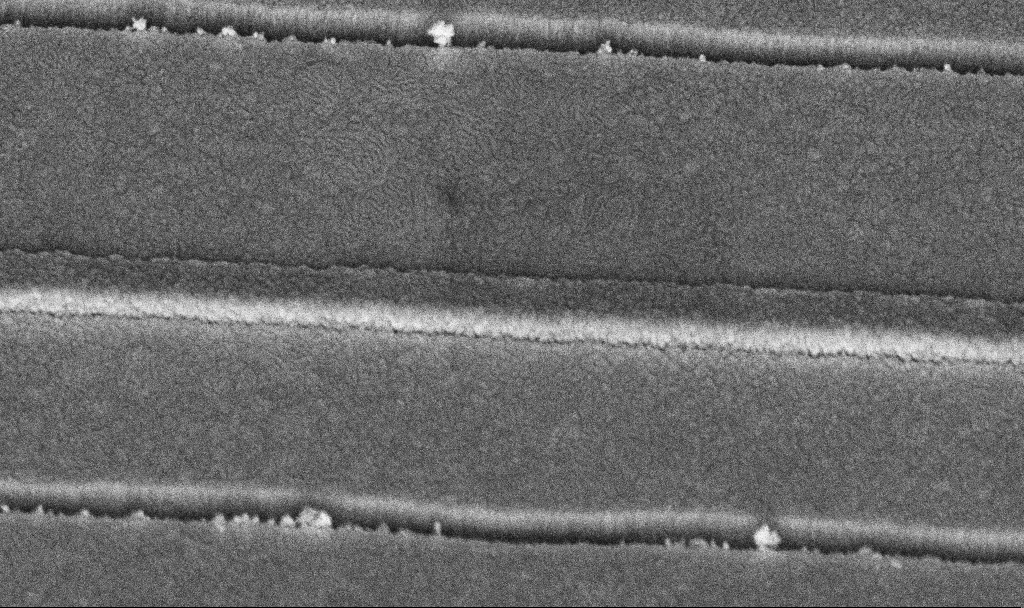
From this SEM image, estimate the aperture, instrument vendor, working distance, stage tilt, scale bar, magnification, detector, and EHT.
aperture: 30 µm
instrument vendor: Zeiss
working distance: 7.5 mm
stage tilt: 45°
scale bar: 200 nm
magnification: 102.8 K X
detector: InLens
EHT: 5 kV